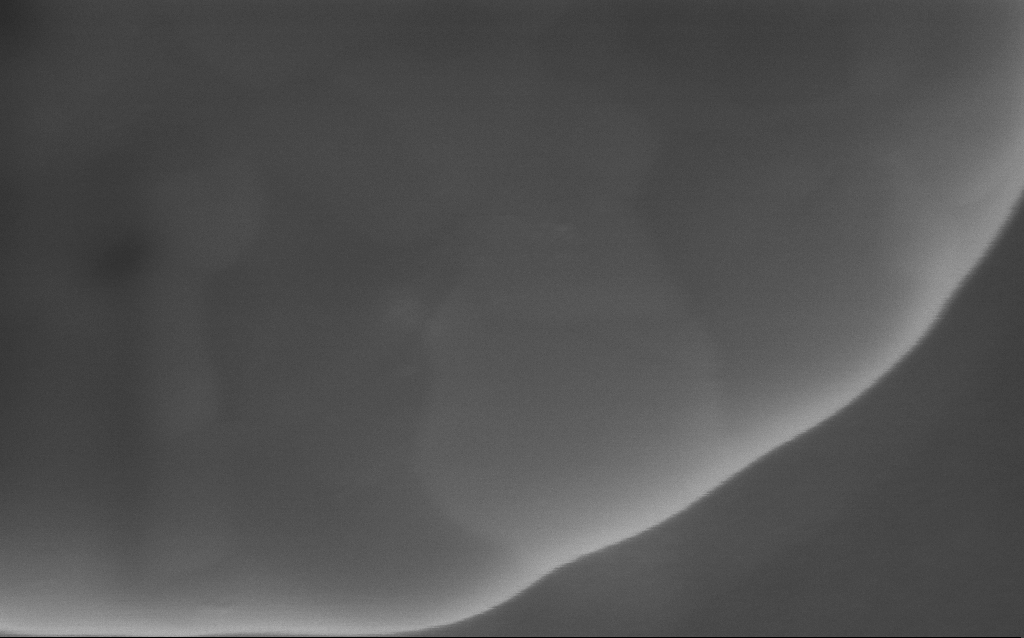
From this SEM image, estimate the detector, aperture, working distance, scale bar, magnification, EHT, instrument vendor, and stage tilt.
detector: InLens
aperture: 30 µm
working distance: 4 mm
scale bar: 200 nm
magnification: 256 K X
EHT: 5 kV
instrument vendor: Zeiss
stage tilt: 0°